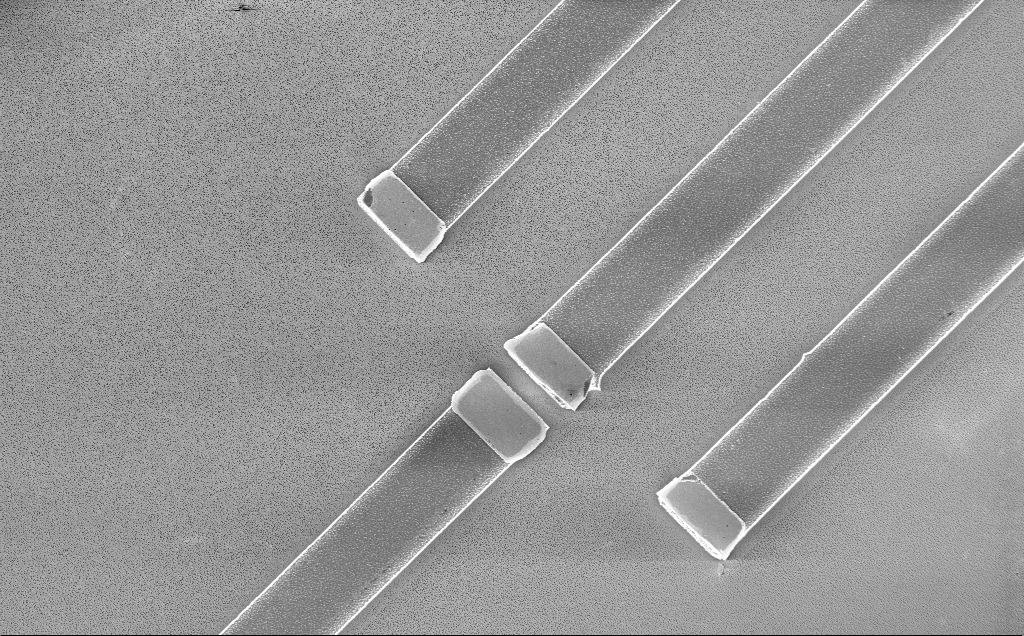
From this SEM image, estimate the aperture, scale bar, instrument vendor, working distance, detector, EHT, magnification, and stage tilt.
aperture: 30 µm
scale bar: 20000 nm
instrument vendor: Zeiss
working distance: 10 mm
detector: InLens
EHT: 2 kV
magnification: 1.95 K X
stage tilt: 0°